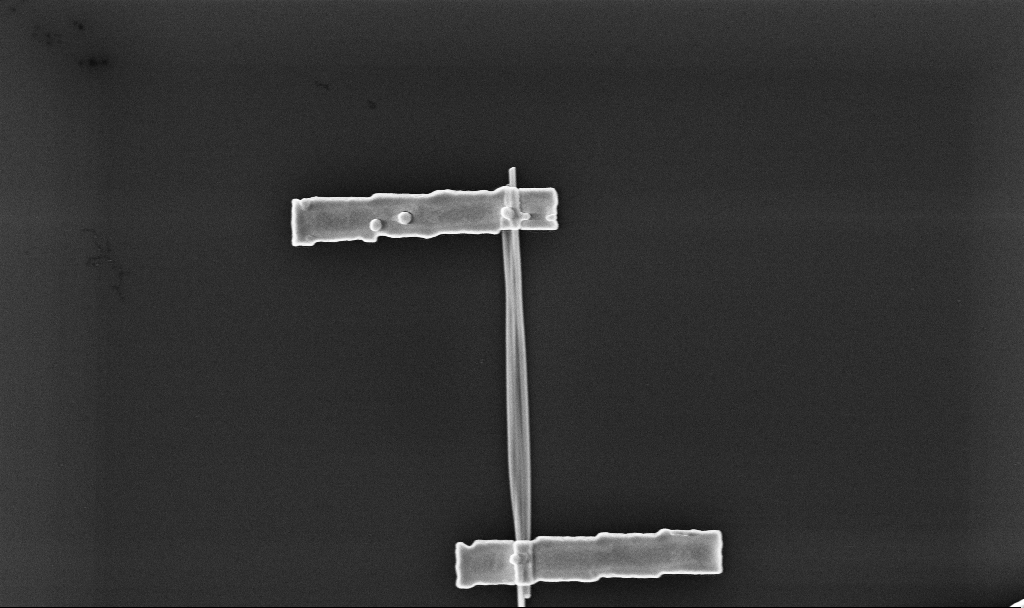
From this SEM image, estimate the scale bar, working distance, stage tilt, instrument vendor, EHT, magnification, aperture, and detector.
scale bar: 2000 nm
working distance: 6.7 mm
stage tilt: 0°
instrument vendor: Zeiss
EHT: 10 kV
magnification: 32.58 K X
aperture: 30 µm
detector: InLens